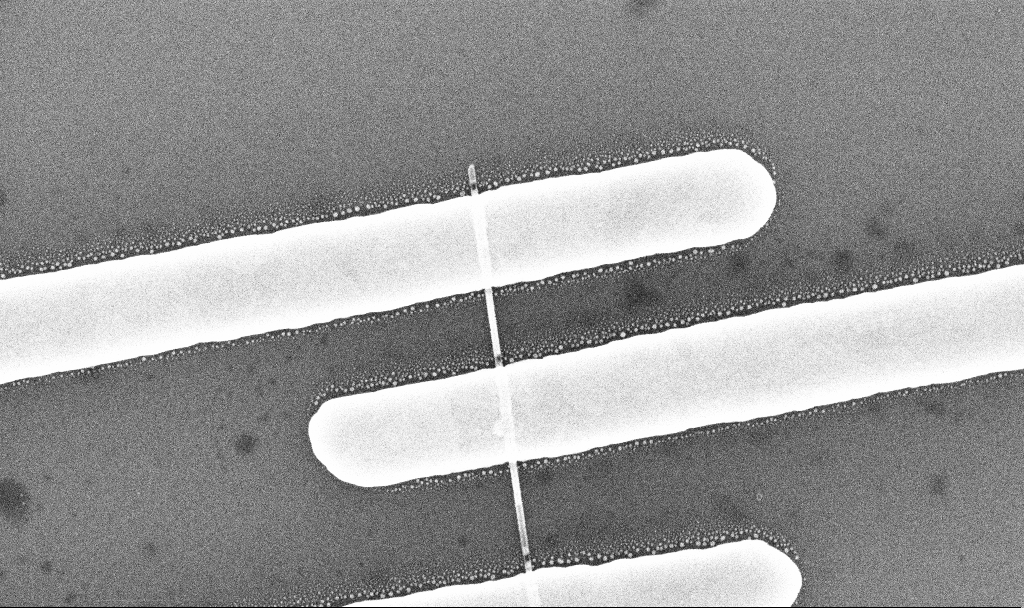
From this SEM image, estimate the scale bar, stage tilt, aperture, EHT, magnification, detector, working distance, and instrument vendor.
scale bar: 200 nm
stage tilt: -0°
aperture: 30 µm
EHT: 10 kV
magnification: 85.22 K X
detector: InLens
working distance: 7 mm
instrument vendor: Zeiss